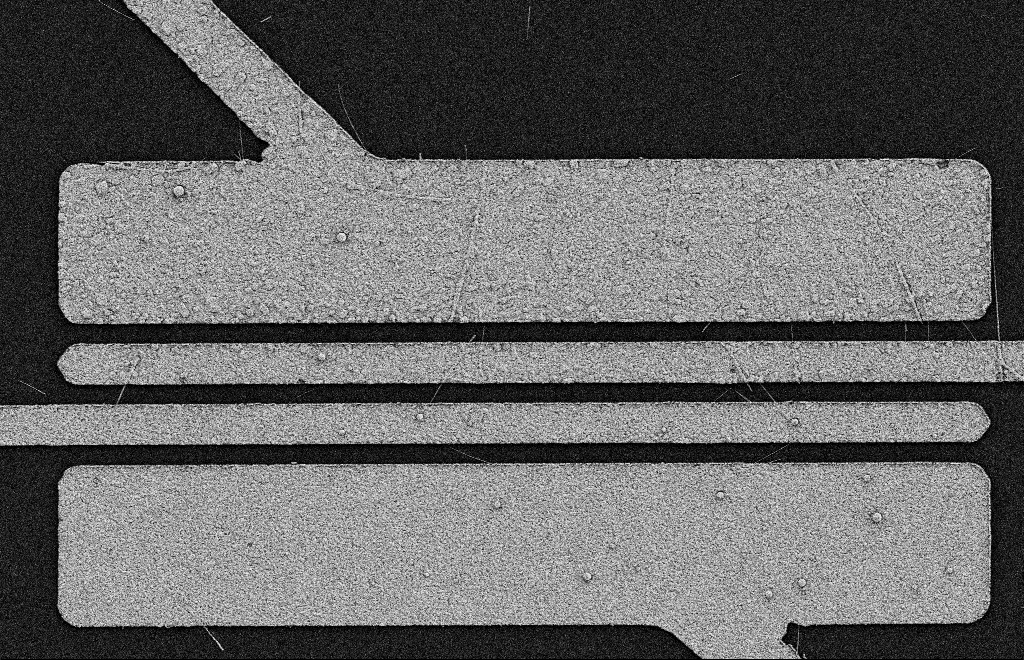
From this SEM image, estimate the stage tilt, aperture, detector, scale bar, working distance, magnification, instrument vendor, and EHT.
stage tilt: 0°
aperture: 20 µm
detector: SE2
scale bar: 2000 nm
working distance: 11 mm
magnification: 5.56 K X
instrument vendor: Zeiss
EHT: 2 kV